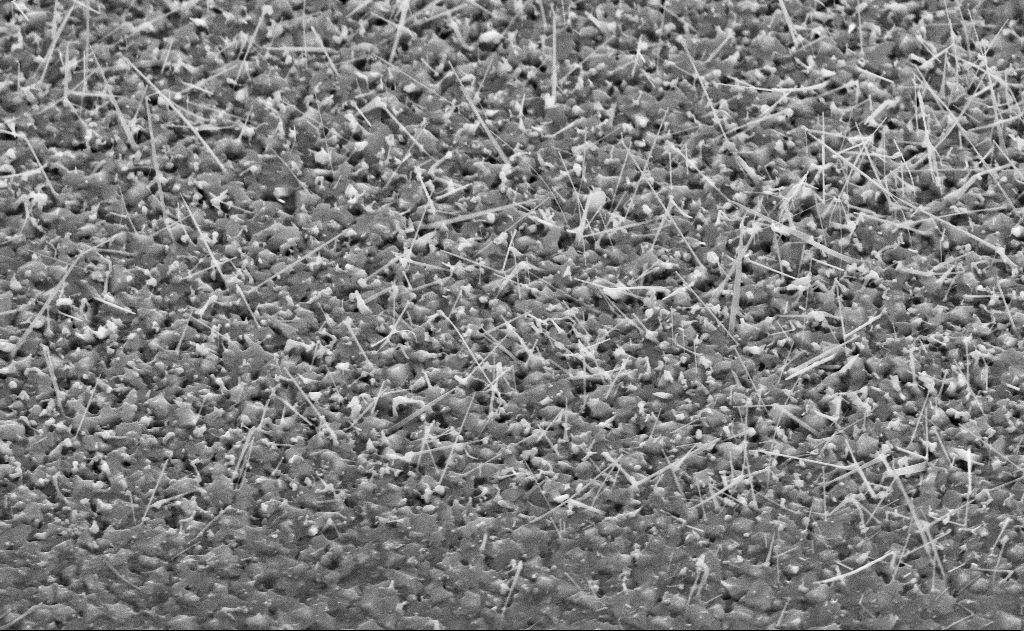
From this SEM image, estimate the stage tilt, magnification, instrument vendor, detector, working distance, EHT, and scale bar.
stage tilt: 45°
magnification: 20 K X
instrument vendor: Zeiss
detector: SE2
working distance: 11 mm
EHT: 10 kV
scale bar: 1000 nm